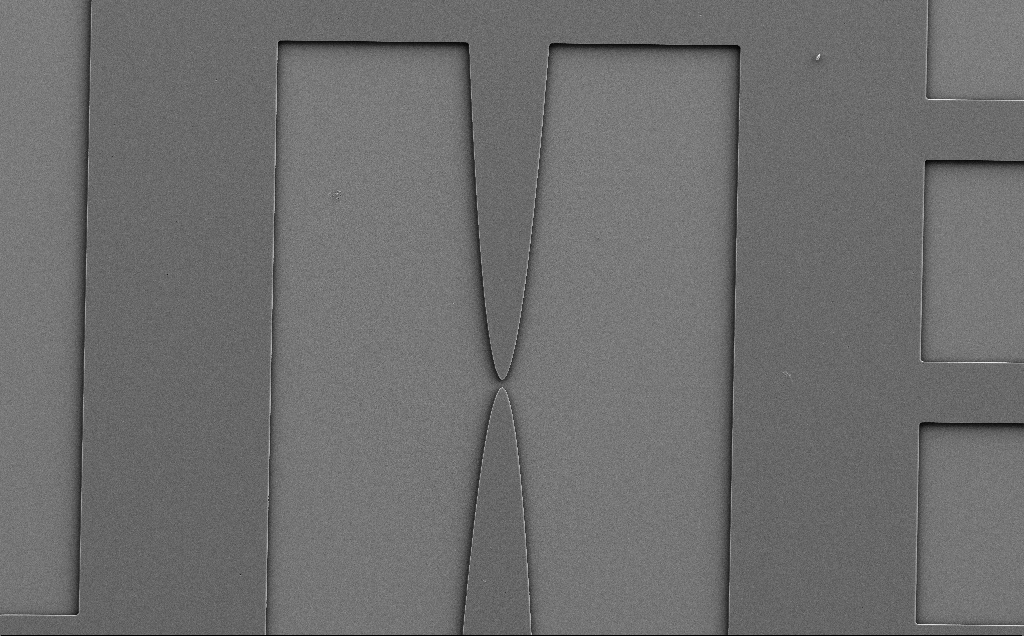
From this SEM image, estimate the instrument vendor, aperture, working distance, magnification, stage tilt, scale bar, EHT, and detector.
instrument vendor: Zeiss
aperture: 30 µm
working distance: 9 mm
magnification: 0.462 K X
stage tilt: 0°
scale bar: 100000 nm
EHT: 5 kV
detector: SE2